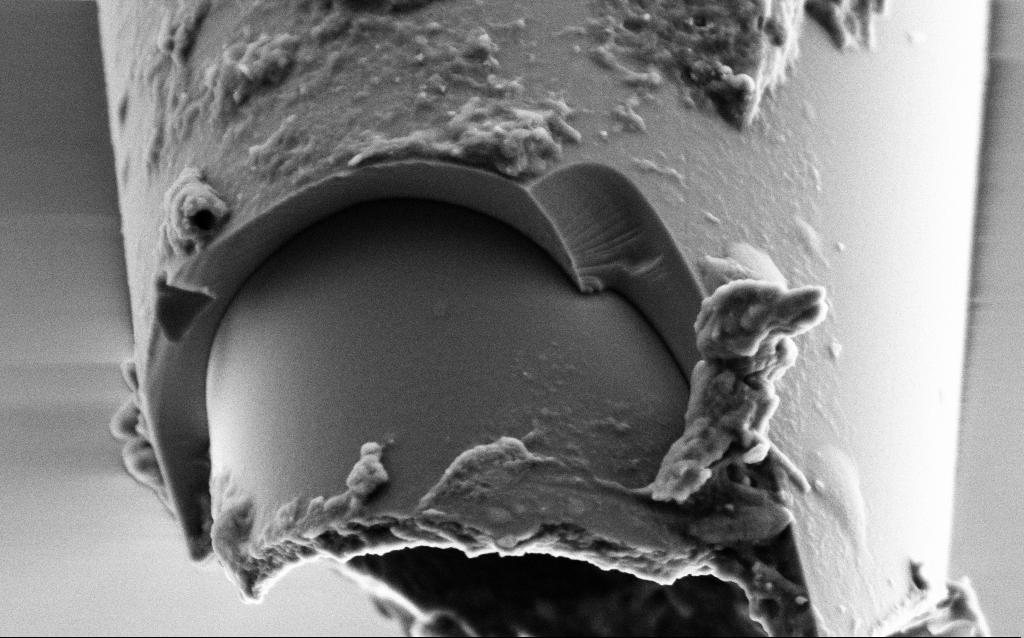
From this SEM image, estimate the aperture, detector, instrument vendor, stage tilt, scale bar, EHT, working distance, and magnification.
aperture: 30 µm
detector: SE2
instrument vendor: Zeiss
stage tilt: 45°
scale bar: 1000 nm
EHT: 2 kV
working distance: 6 mm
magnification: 50 K X